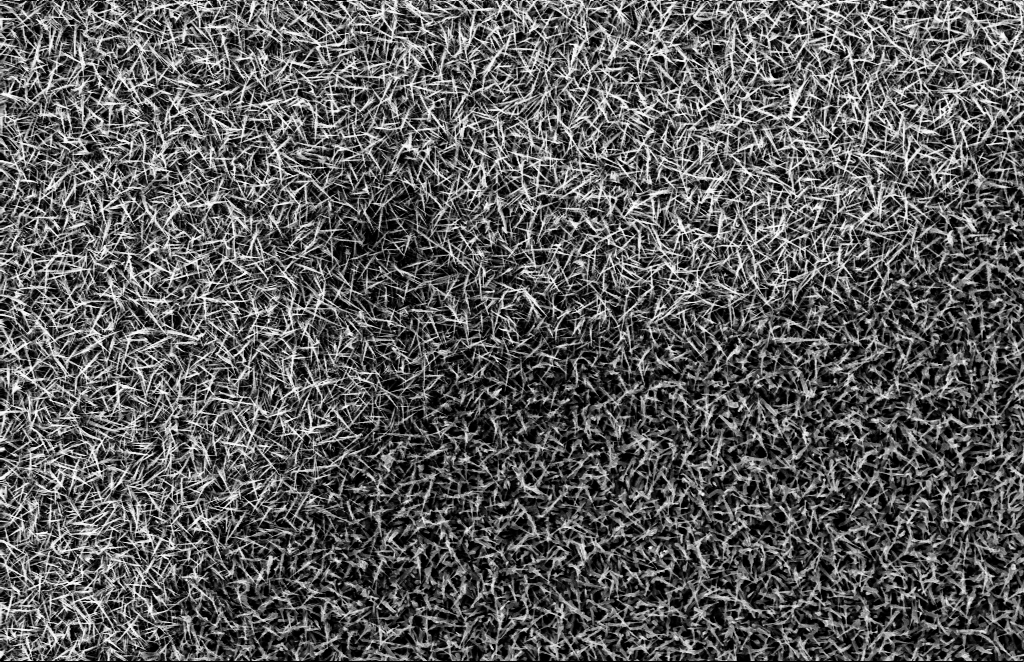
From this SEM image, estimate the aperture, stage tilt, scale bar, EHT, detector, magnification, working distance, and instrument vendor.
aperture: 30 µm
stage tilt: -1.1°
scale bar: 2000 nm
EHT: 10 kV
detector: InLens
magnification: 10 K X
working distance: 10 mm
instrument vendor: Zeiss